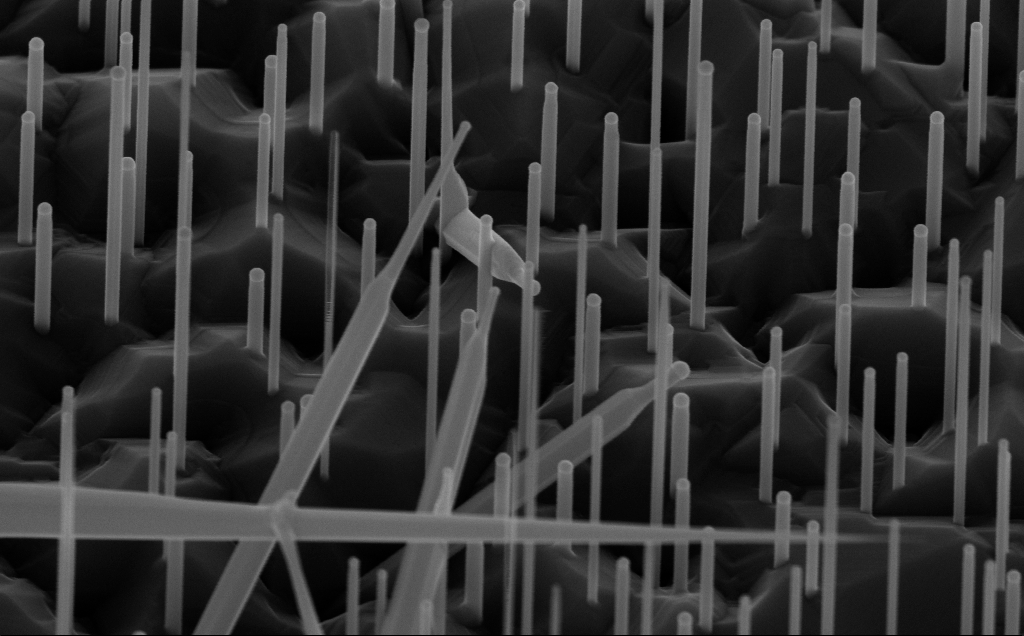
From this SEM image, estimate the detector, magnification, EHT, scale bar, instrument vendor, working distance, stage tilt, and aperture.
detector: InLens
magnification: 80 K X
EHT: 10 kV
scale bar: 200 nm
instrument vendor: Zeiss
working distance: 7 mm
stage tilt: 45°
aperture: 30 µm